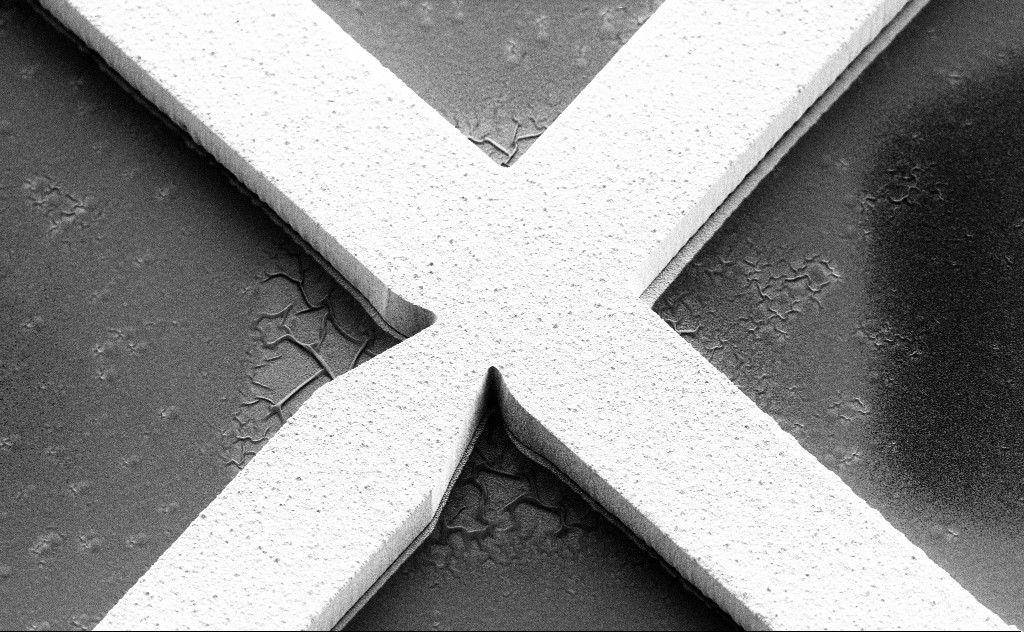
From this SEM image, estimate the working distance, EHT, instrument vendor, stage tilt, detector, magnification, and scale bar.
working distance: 11 mm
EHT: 3 kV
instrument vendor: Zeiss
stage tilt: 45°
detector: SE2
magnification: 5.55 K X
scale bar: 10000 nm